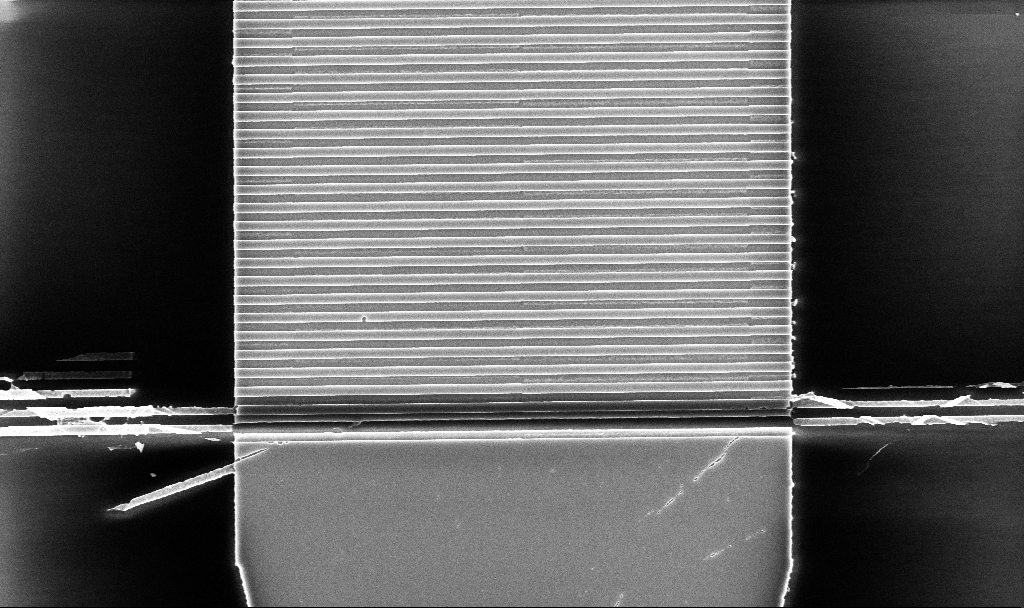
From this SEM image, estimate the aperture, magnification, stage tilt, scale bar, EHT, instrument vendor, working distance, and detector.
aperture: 30 µm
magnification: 10.37 K X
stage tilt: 0°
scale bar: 2000 nm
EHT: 5 kV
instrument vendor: Zeiss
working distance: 5.2 mm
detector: InLens